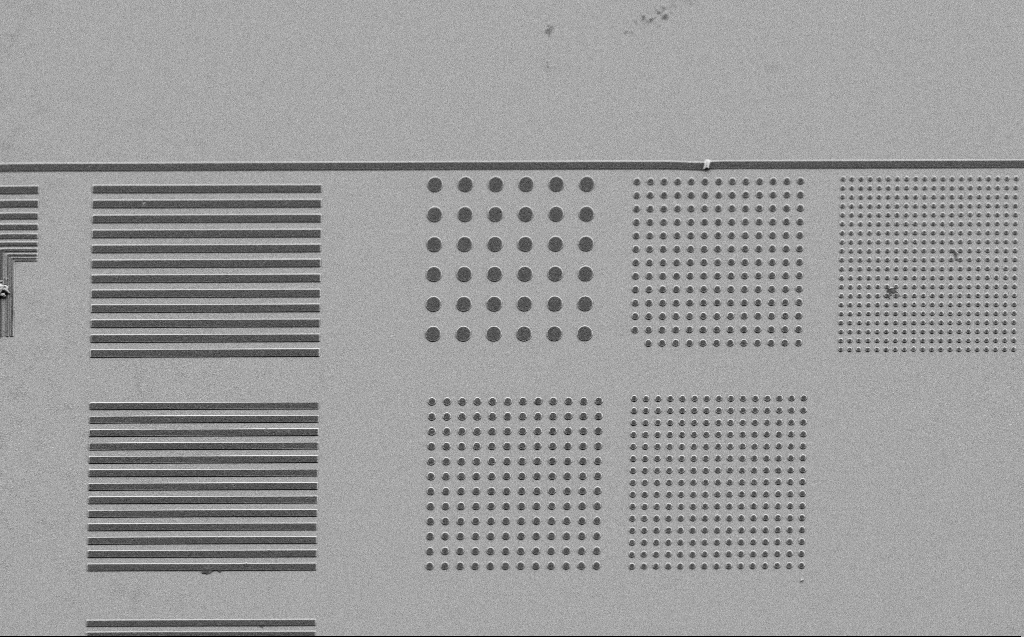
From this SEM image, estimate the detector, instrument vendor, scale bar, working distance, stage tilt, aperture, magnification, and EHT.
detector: SE2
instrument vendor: Zeiss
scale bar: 10000 nm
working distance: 5 mm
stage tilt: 30°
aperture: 30 µm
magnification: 2.81 K X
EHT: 2.5 kV